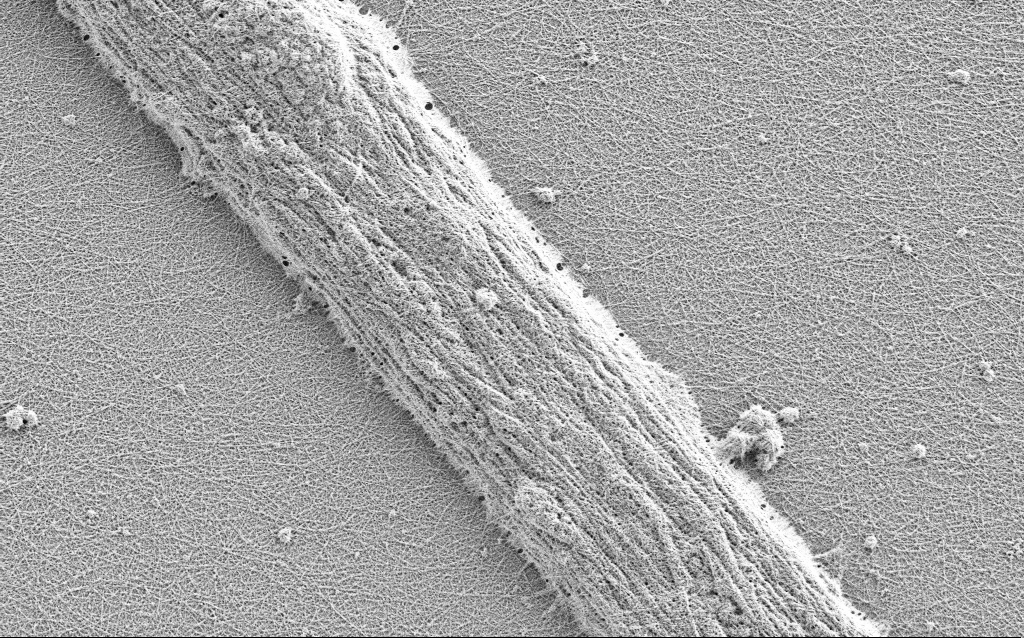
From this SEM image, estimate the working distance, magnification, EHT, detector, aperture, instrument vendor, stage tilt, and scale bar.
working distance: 3 mm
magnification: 10 K X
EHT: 0.9 kV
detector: SE2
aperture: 30 µm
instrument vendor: Zeiss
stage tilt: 0°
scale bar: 2000 nm